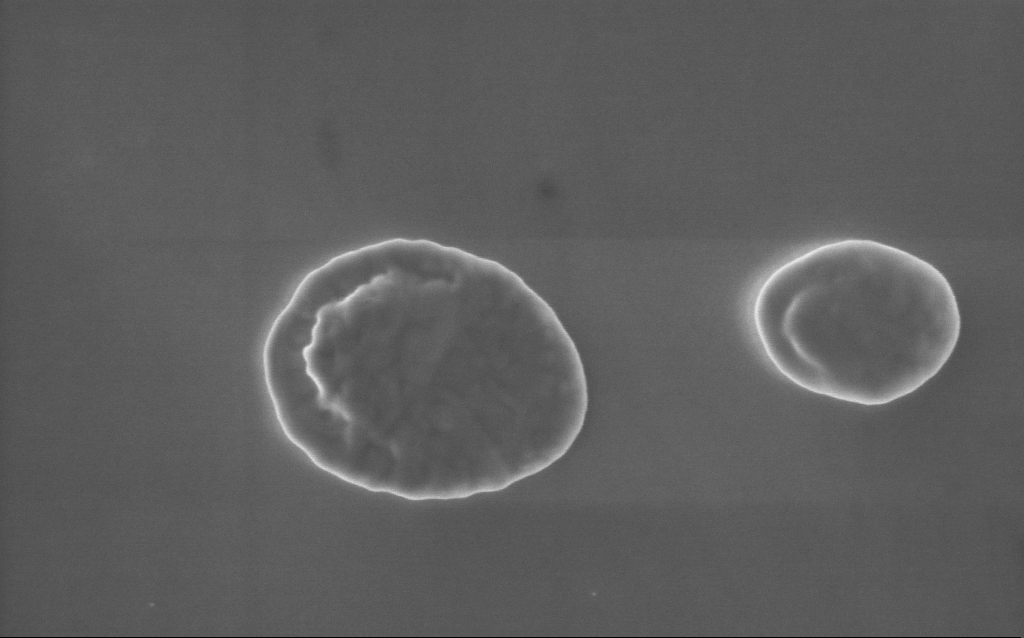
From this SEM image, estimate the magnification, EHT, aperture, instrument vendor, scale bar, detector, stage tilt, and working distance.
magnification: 58 K X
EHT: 5 kV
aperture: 30 µm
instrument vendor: Zeiss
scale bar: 1000 nm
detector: InLens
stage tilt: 0°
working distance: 3 mm